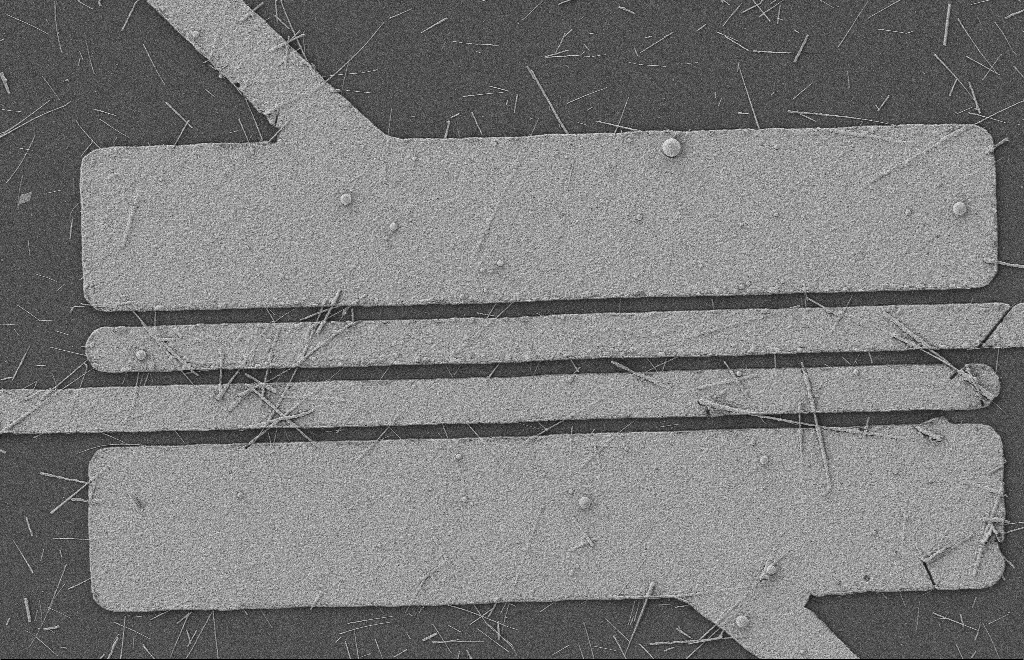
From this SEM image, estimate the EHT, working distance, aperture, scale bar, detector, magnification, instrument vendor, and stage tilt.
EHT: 2 kV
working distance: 8 mm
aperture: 20 µm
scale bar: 2000 nm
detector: SE2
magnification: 5.48 K X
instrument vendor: Zeiss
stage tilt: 0°